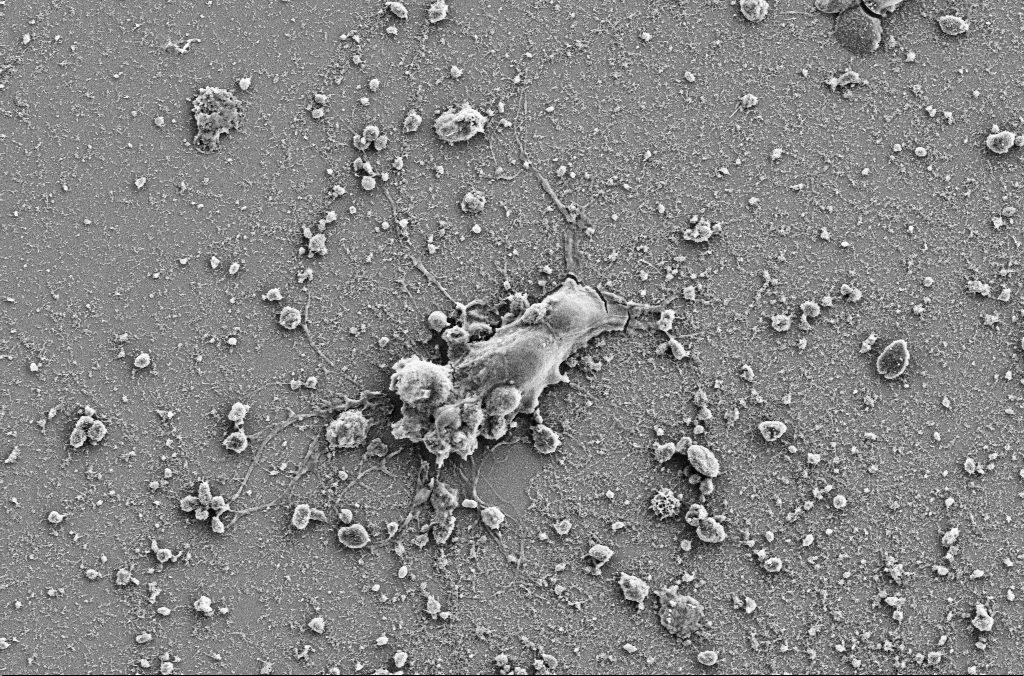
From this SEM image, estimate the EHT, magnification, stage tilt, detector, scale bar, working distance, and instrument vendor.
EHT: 5 kV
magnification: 3 K X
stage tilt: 0°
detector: SE2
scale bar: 10000 nm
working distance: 4 mm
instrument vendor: Zeiss